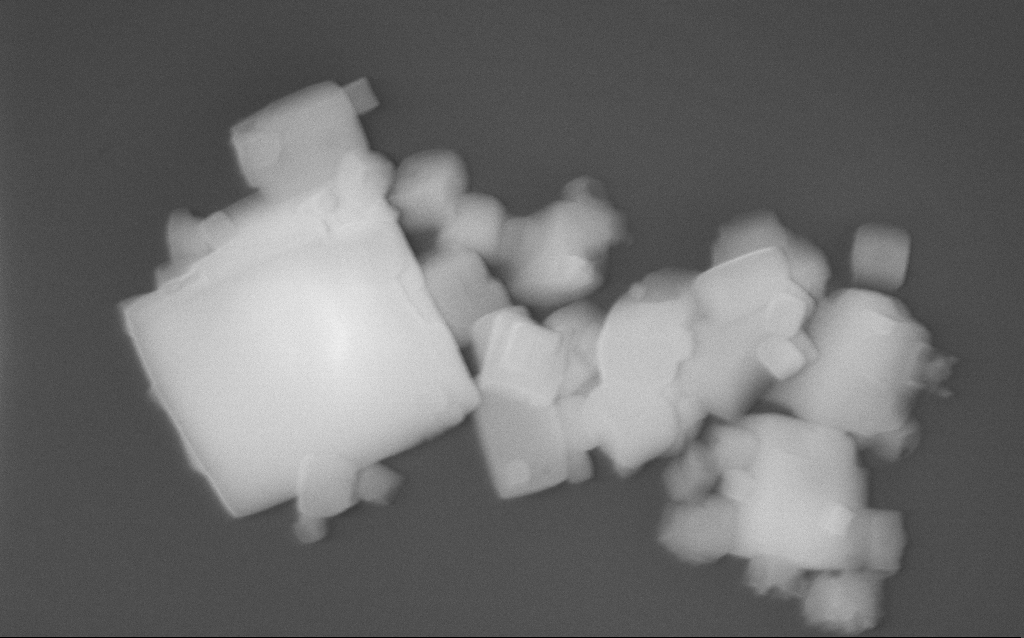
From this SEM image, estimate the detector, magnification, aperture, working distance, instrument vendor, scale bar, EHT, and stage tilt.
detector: InLens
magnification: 56.3 K X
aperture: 30 µm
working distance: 3 mm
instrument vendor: Zeiss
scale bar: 1000 nm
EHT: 10 kV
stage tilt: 0°